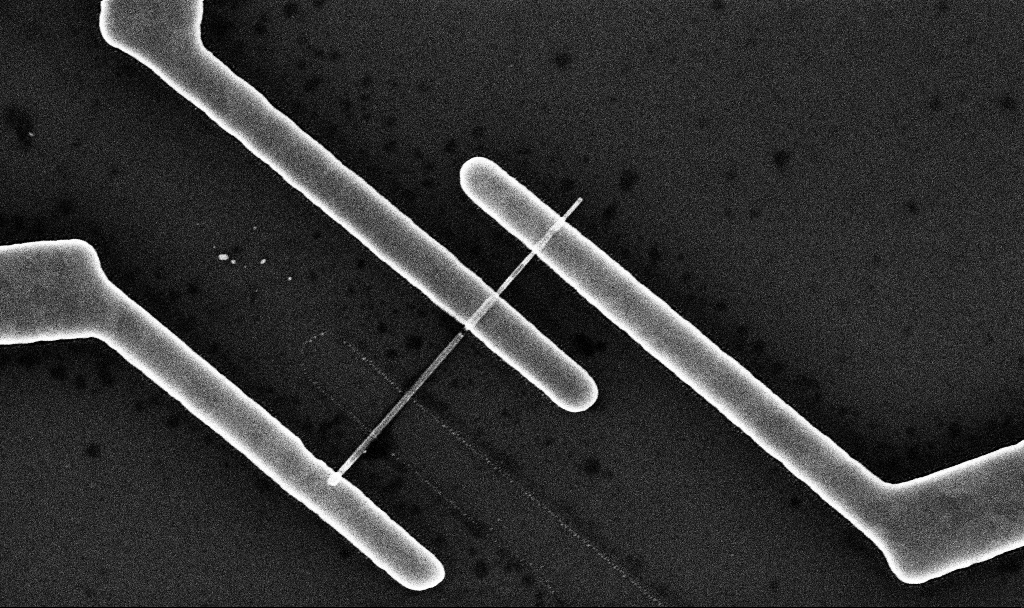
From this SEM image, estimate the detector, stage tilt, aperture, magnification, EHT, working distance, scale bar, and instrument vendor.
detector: InLens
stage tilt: -0°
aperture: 30 µm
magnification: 42.46 K X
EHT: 10 kV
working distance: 7 mm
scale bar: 1000 nm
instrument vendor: Zeiss